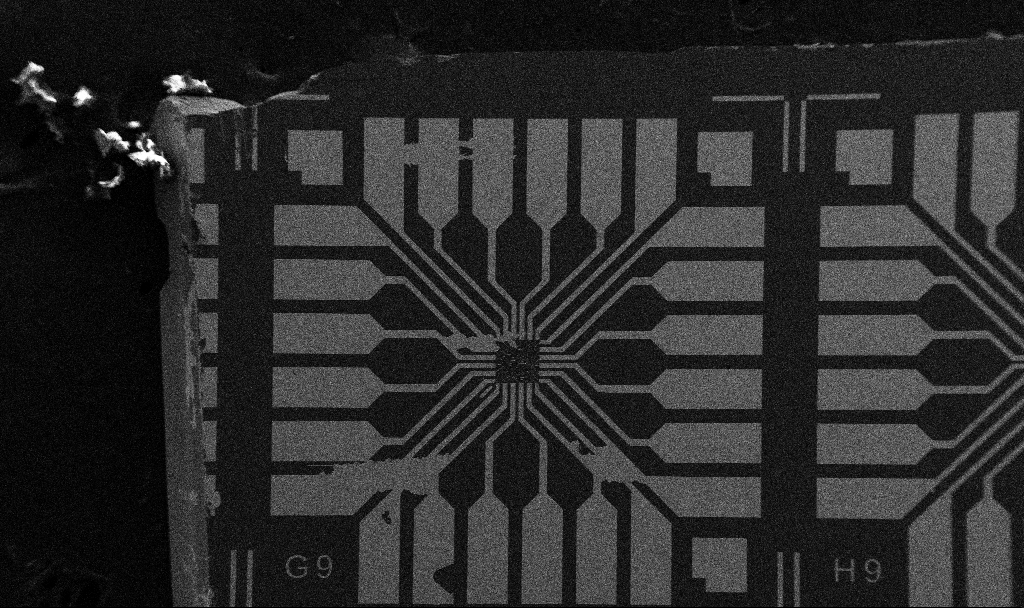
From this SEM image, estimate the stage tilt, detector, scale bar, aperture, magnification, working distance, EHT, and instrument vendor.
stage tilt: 0°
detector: SE2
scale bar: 200000 nm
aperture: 30 µm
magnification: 0.1 K X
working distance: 10.7 mm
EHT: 5 kV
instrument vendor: Zeiss